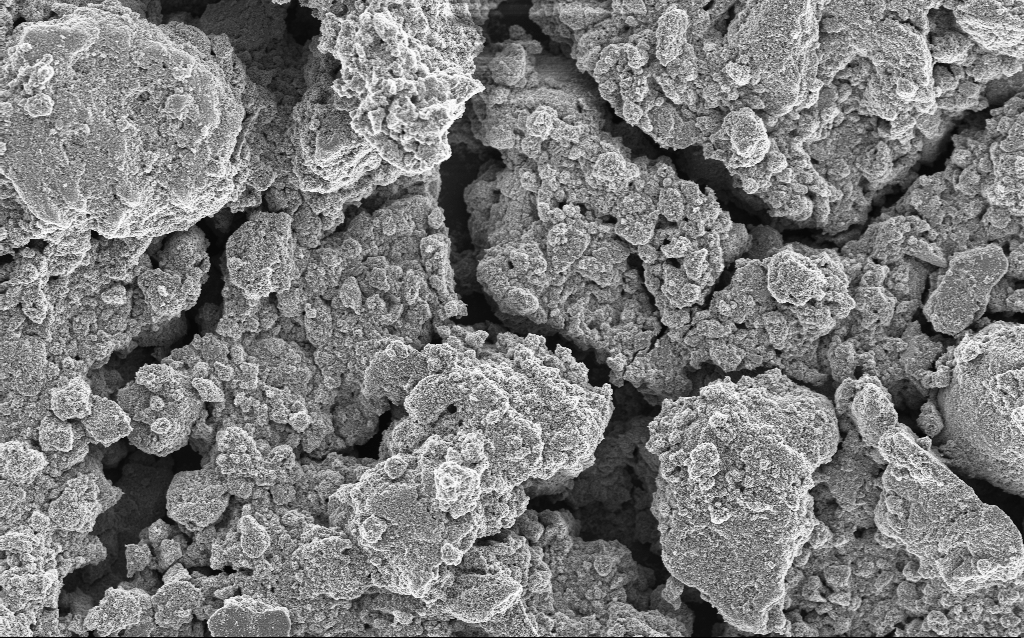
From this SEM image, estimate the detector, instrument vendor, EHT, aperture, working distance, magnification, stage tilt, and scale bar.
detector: InLens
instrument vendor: Zeiss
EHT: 5 kV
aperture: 30 µm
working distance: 4.5 mm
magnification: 1.23 K X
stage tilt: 0°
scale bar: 10000 nm